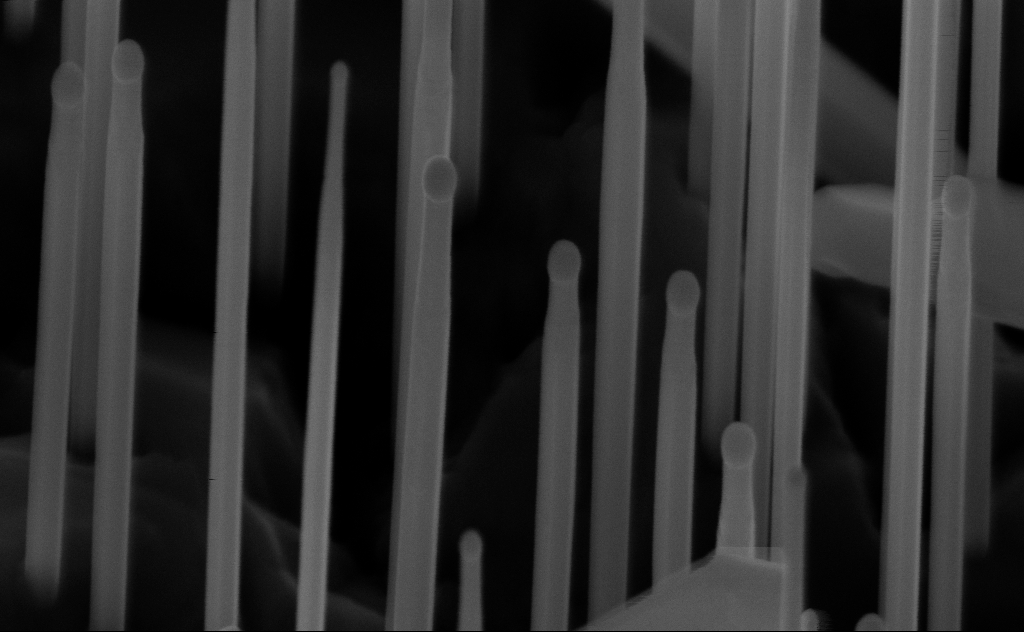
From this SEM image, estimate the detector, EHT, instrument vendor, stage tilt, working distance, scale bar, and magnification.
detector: InLens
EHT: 10 kV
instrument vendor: Zeiss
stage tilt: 45°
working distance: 6 mm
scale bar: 200 nm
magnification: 165.64 K X